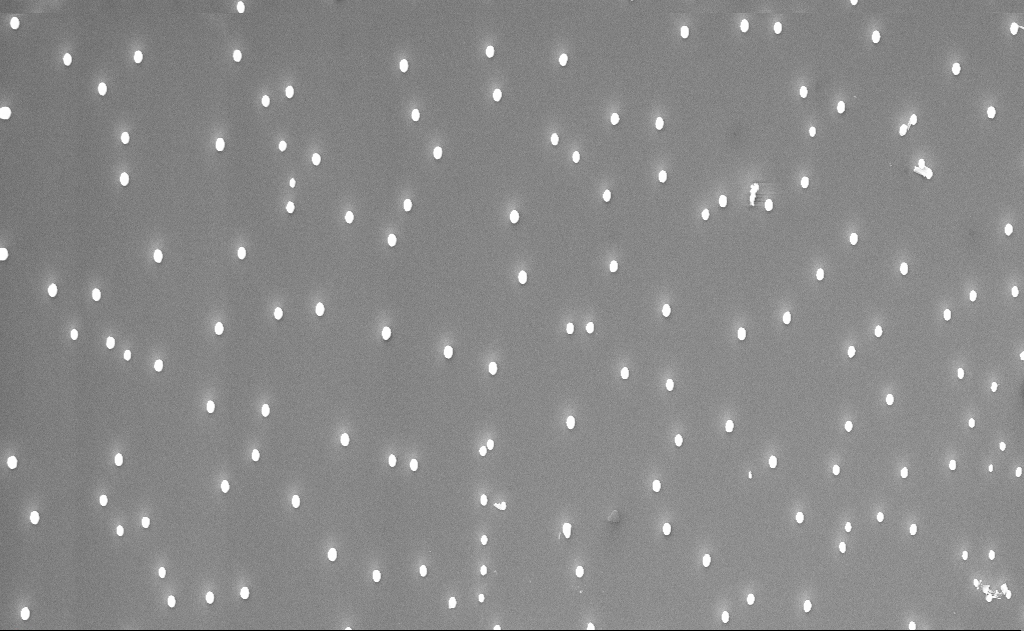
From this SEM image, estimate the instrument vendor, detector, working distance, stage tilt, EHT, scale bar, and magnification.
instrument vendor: Zeiss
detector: InLens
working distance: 12 mm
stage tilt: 0°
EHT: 10 kV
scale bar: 2000 nm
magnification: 10 K X